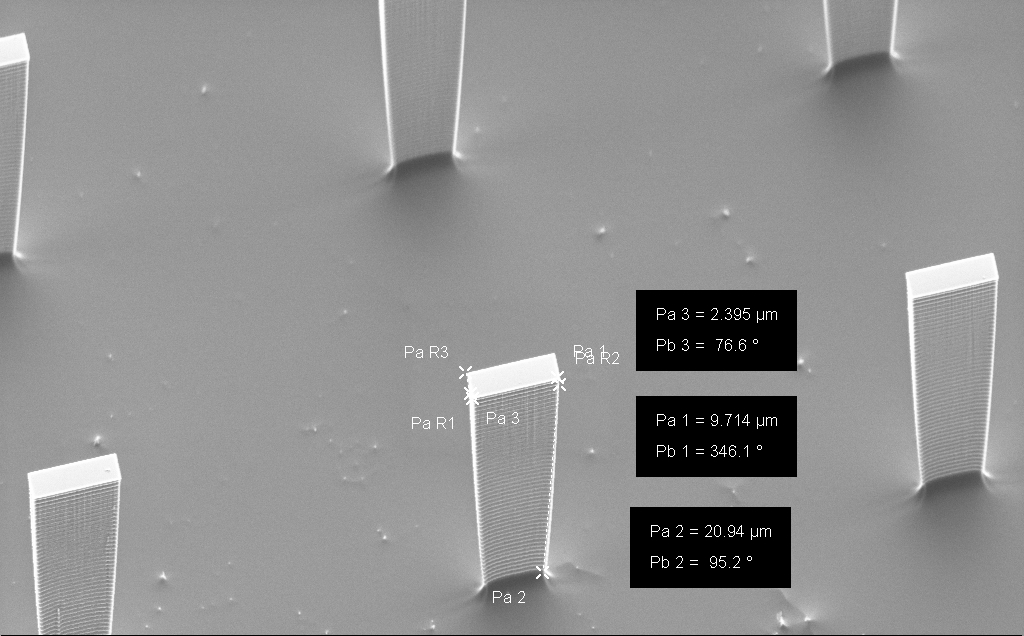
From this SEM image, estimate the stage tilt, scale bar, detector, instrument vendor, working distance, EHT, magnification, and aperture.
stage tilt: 50°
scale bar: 10000 nm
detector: SE2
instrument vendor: Zeiss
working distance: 16 mm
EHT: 5 kV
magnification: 3.31 K X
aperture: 30 µm